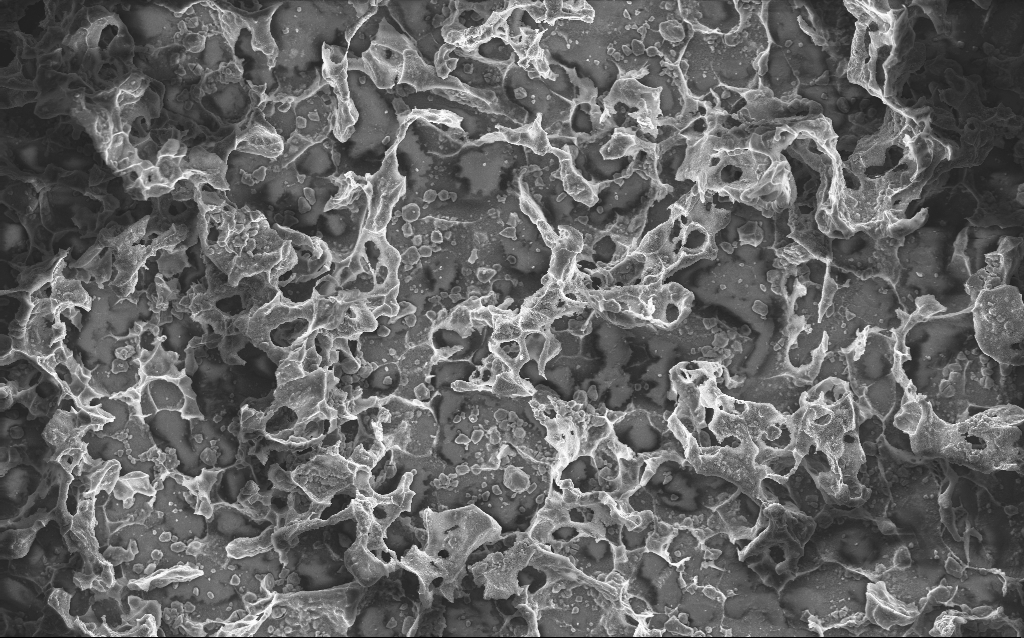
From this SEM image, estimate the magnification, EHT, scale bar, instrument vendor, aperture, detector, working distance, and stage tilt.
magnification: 0.792 K X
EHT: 10 kV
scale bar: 20000 nm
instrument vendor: Zeiss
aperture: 30 µm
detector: InLens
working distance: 2.7 mm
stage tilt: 0°